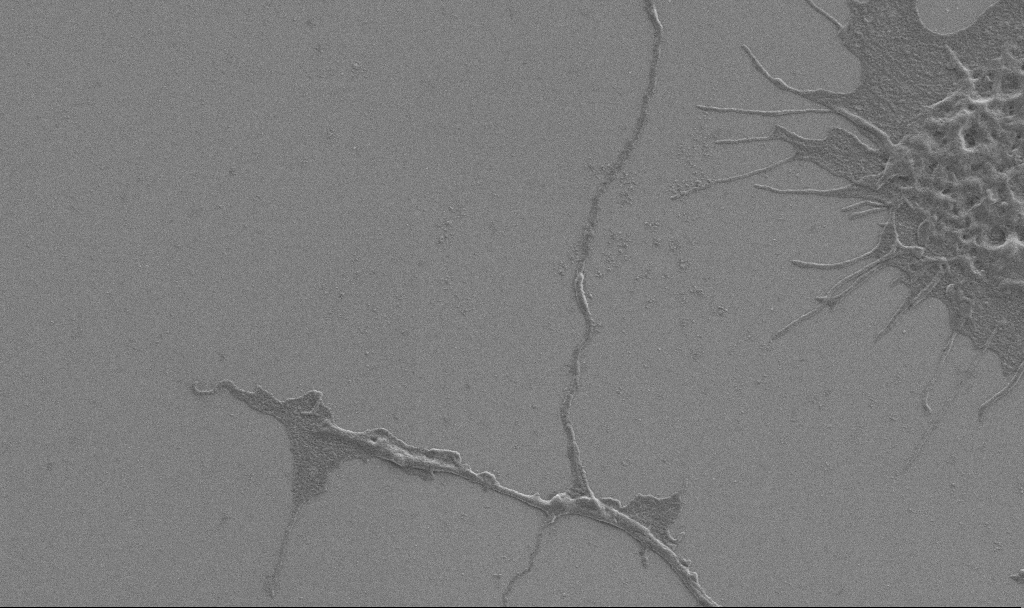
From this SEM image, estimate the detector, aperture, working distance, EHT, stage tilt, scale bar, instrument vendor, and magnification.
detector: SE2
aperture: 30 µm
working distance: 6.9 mm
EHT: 0.9 kV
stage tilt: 0°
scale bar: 2000 nm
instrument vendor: Zeiss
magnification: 10 K X